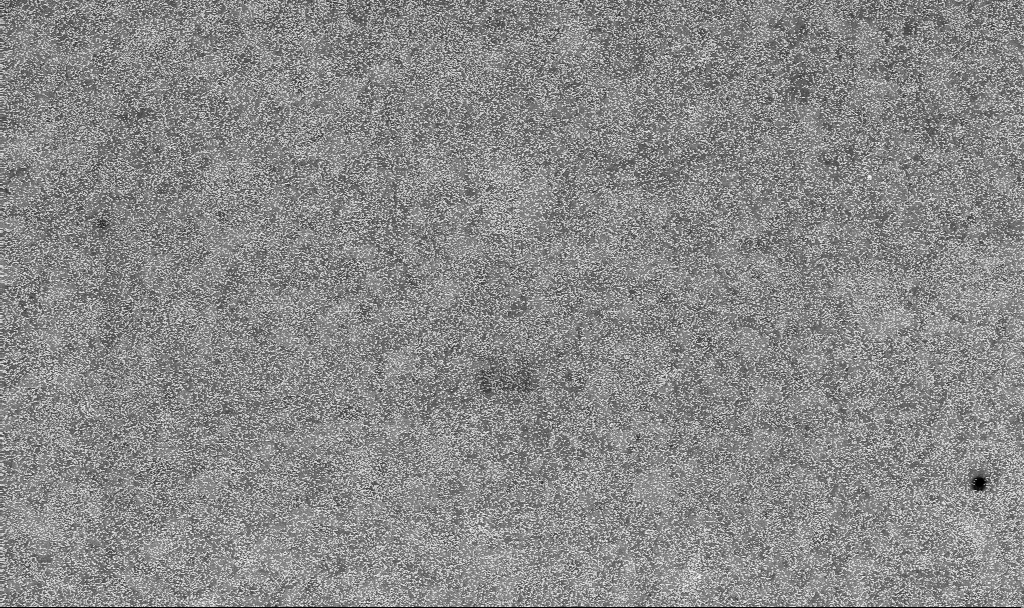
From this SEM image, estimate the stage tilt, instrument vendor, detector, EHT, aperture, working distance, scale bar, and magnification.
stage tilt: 0°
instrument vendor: Zeiss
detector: InLens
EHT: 10 kV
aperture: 30 µm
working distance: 3.3 mm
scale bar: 1000 nm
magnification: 20 K X